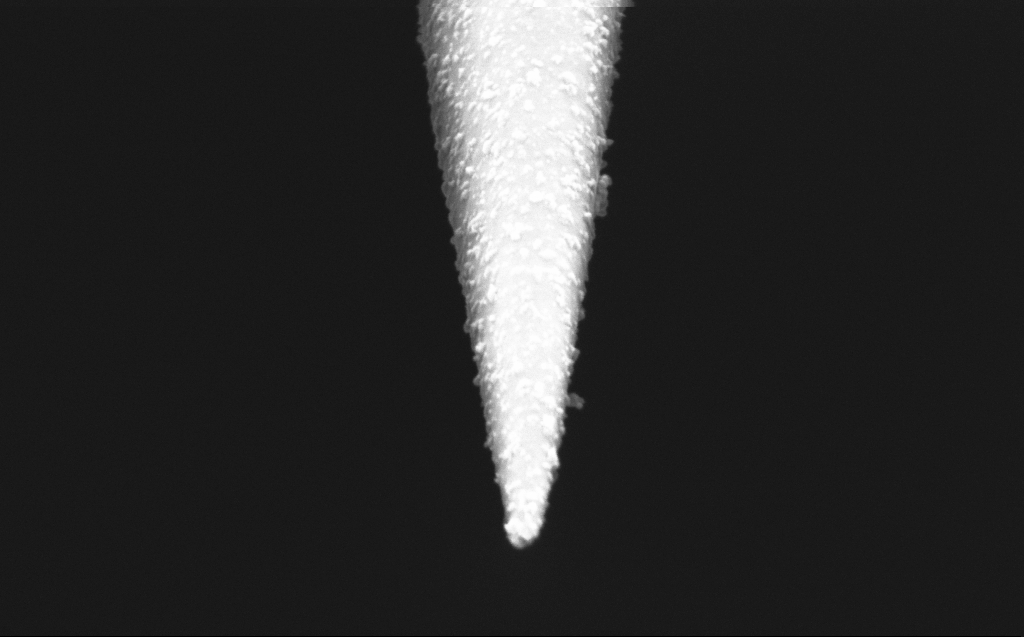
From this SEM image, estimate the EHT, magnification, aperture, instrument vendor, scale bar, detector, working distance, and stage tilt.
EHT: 2 kV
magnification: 100 K X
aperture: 30 µm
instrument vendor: Zeiss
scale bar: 200 nm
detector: InLens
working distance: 4 mm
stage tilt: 45°